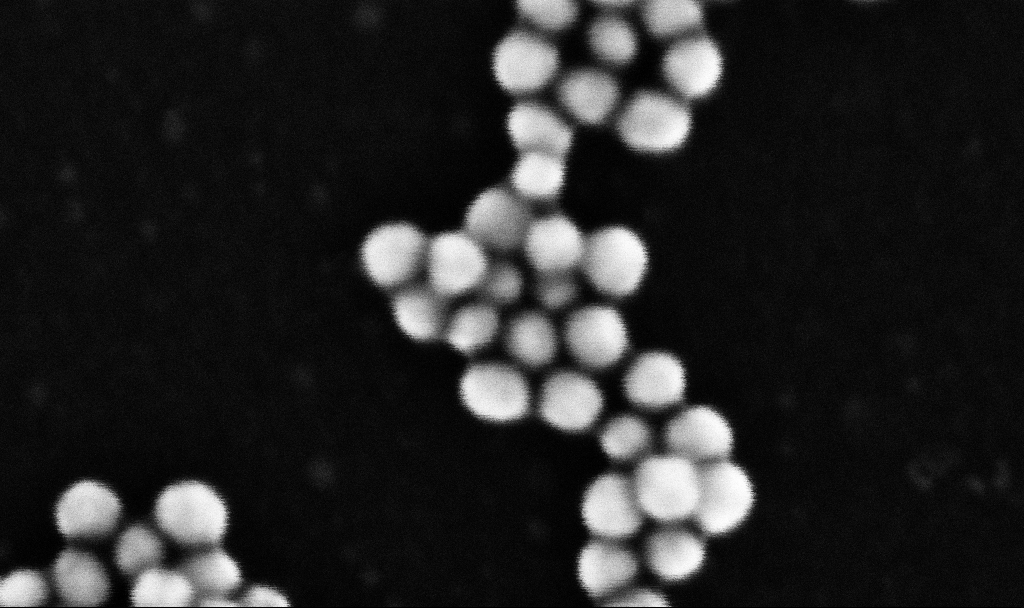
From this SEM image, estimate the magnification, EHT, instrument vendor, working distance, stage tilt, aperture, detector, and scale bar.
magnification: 1100.44 K X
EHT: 10 kV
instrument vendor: Zeiss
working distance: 3.2 mm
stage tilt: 0°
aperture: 30 µm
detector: InLens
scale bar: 20 nm